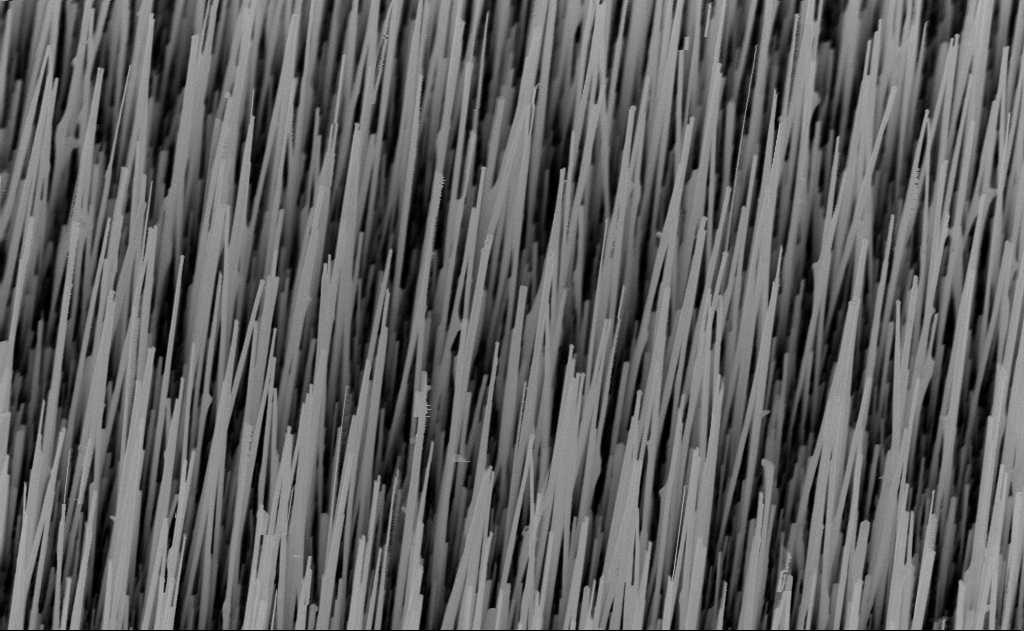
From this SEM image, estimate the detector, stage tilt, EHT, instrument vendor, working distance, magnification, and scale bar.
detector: InLens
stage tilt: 30°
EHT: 10 kV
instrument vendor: Zeiss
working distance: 9 mm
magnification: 20 K X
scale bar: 2000 nm